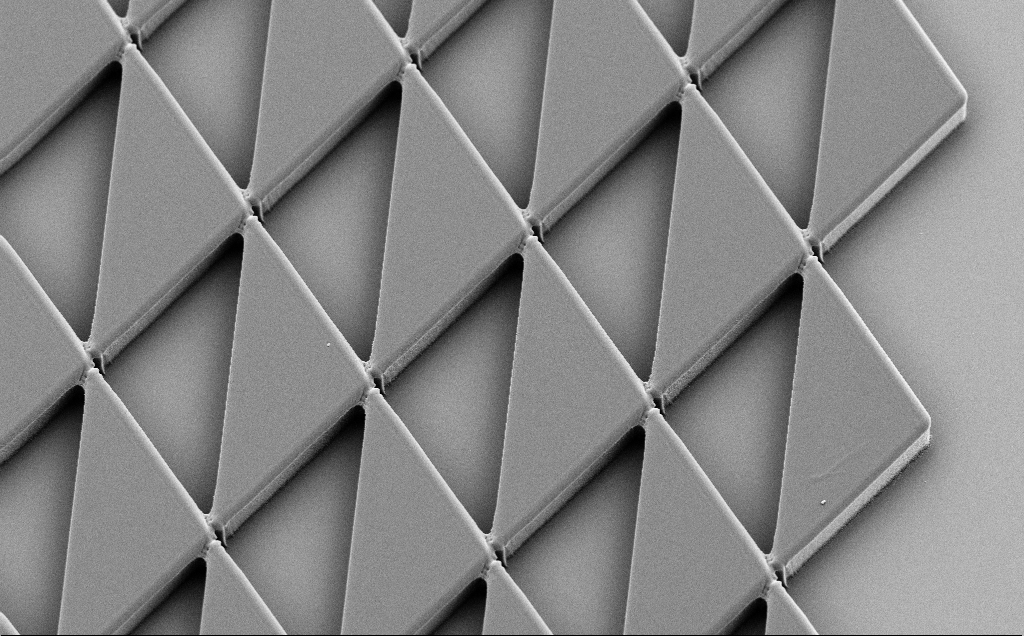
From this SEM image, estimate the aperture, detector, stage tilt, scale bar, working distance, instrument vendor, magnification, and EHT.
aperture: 30 µm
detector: SE2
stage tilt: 30°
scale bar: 20000 nm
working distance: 8 mm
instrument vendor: Zeiss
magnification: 1.47 K X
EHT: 5 kV